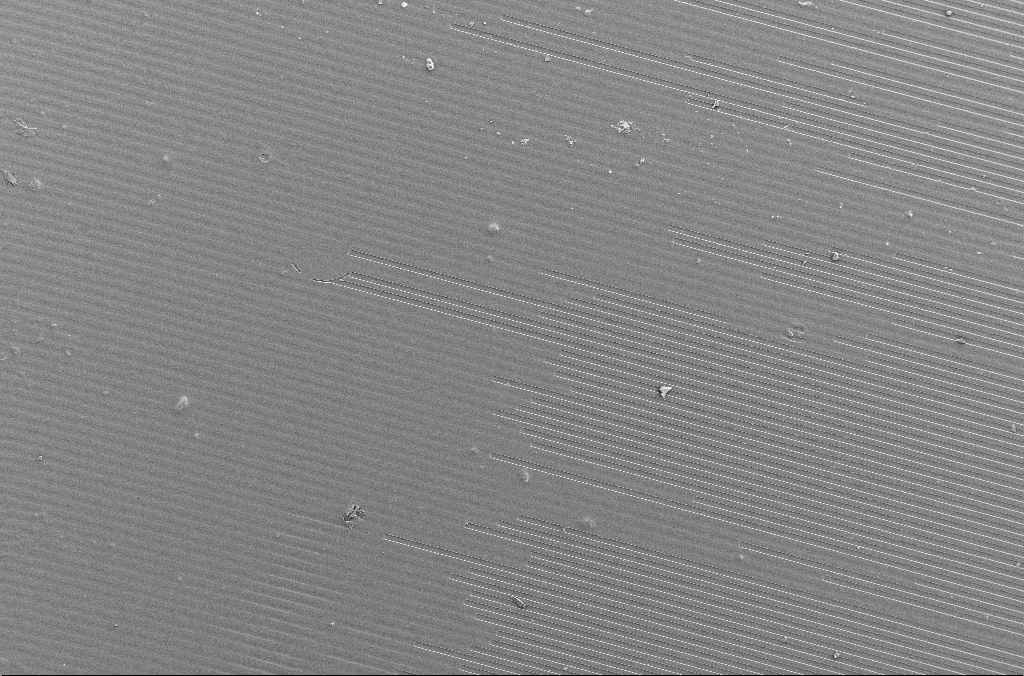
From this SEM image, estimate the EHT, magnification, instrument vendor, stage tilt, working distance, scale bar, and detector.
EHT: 5 kV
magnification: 0.2 K X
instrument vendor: Zeiss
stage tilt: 0°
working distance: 4.1 mm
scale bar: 100000 nm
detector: SE2